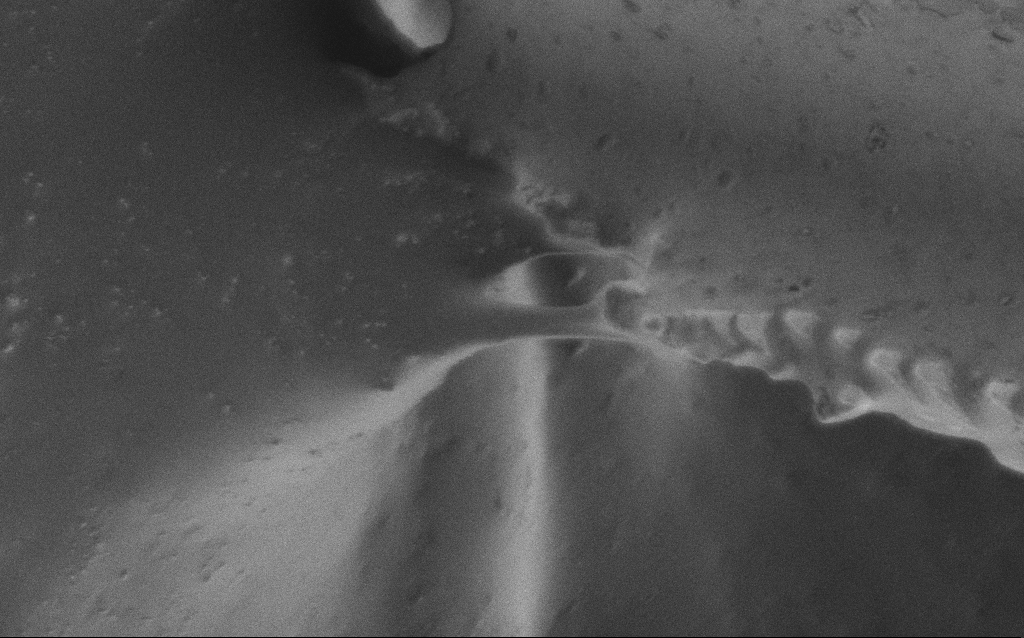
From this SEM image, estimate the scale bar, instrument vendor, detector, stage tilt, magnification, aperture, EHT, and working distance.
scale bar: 2000 nm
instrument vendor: Zeiss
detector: SE2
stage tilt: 0°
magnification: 13.99 K X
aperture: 30 µm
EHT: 1 kV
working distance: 5 mm